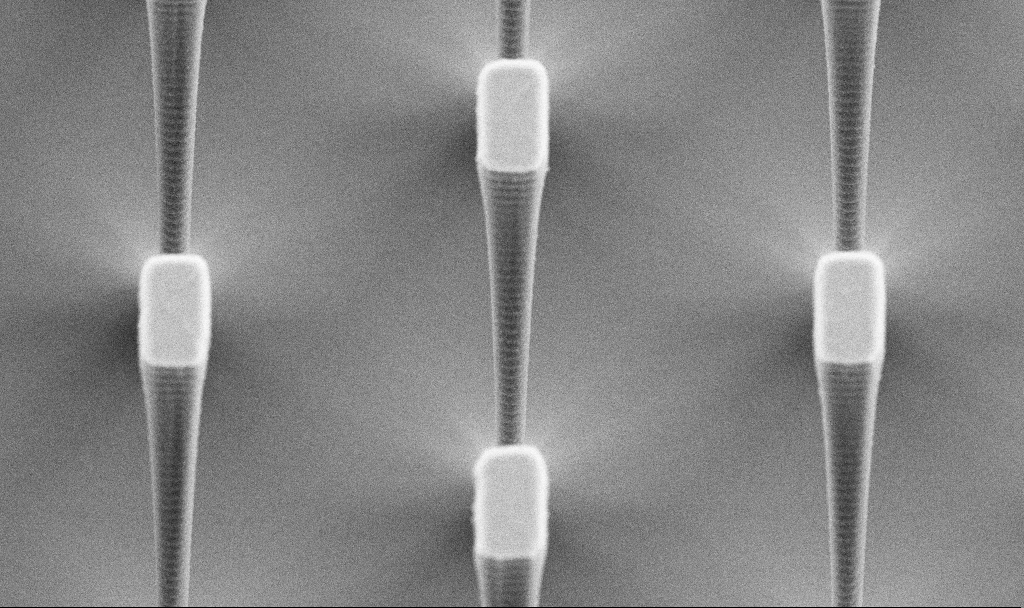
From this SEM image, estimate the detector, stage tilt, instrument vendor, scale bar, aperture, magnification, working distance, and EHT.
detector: SE2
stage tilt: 30°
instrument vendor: Zeiss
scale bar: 2000 nm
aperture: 30 µm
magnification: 13.75 K X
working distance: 6.9 mm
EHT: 5 kV